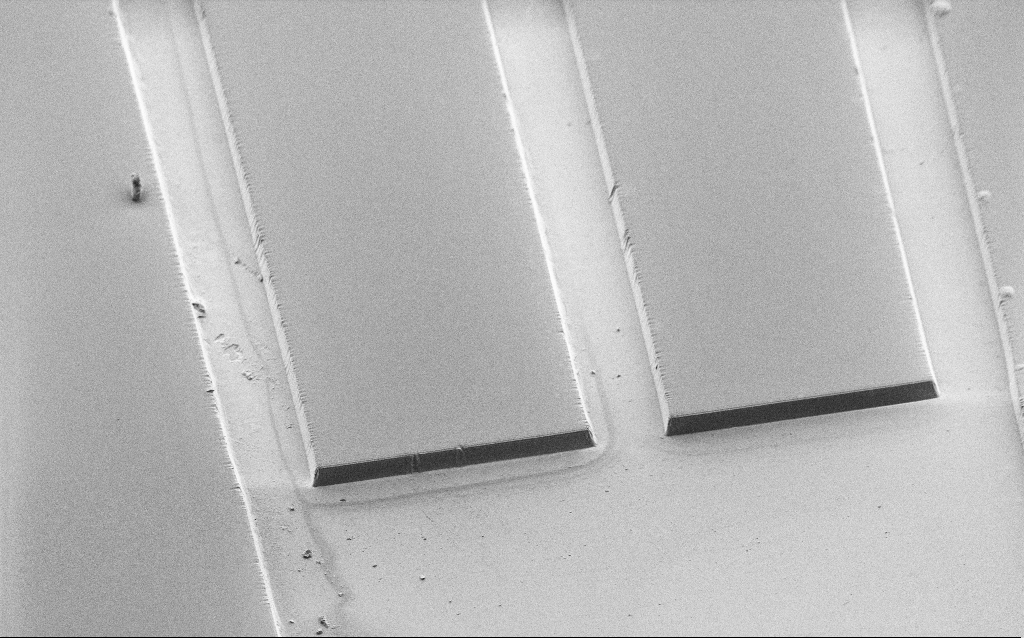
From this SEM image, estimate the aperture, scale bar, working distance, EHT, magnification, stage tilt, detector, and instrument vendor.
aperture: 30 µm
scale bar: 20000 nm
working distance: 8 mm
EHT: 1.2 kV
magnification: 1.22 K X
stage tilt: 45°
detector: SE2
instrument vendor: Zeiss